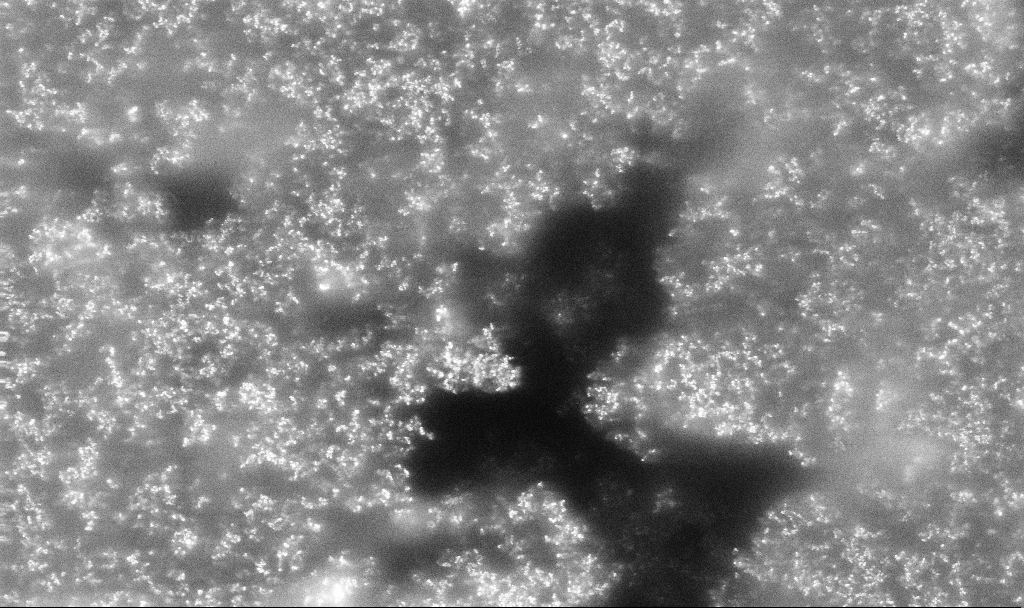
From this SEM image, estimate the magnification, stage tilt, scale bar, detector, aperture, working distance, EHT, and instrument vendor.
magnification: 8.99 K X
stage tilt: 0°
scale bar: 2000 nm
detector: InLens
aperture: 30 µm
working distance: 2.4 mm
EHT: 10 kV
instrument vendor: Zeiss